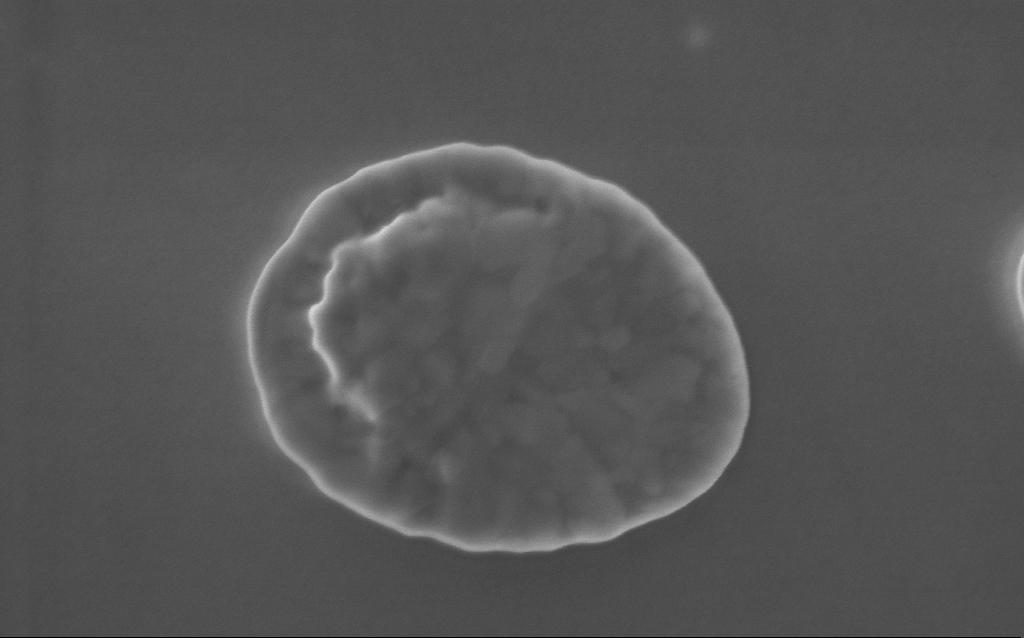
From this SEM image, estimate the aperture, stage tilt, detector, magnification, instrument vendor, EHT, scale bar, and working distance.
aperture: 30 µm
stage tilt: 0°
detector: InLens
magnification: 90.7 K X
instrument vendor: Zeiss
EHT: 5 kV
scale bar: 200 nm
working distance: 3 mm